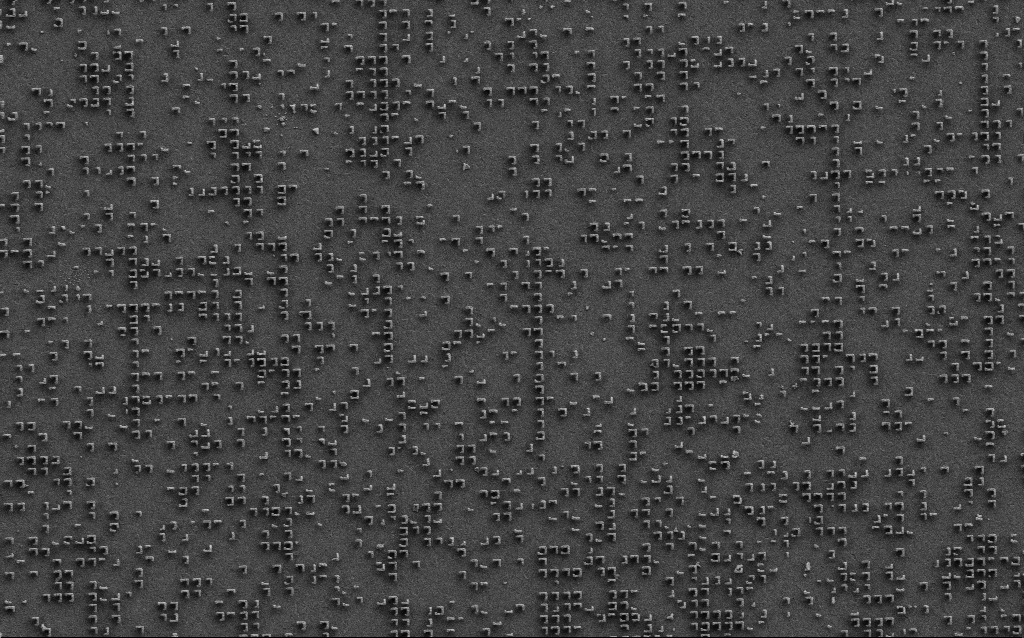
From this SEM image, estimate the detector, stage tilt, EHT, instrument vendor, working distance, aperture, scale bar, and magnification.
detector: SE2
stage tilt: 0°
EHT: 3 kV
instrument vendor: Zeiss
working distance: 6.7 mm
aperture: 30 µm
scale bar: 2000 nm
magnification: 9.21 K X